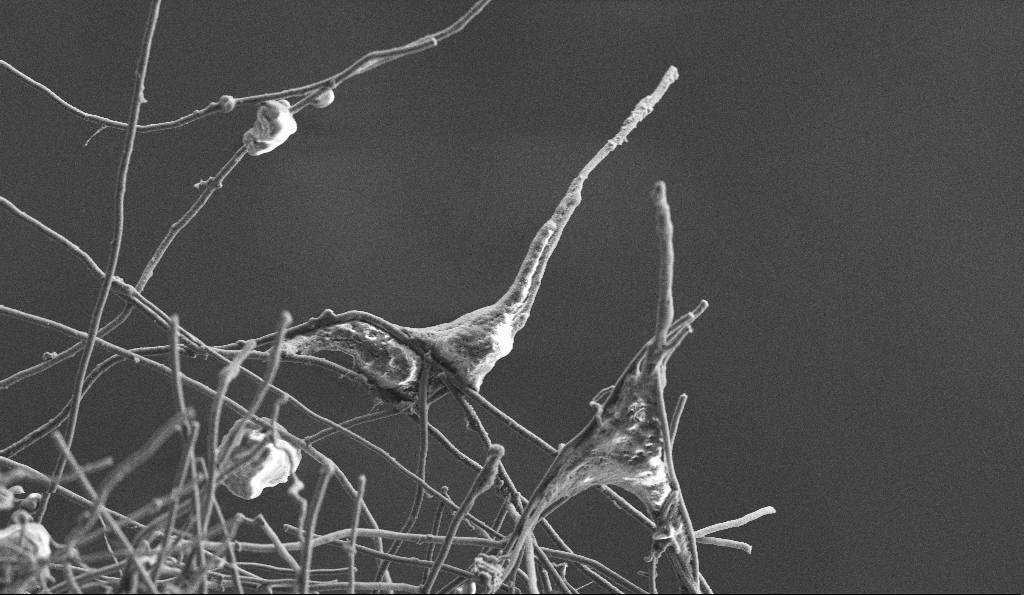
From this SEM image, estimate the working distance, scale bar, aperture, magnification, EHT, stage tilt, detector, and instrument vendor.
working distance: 5.9 mm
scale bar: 10000 nm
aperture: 30 µm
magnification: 5 K X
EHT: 3 kV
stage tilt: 0°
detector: SE2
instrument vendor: Zeiss